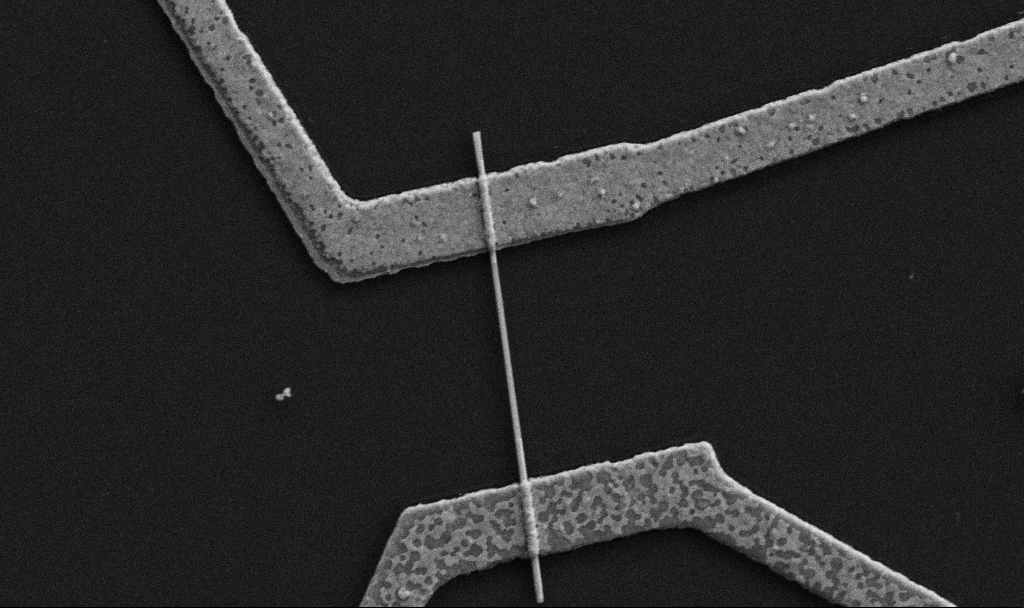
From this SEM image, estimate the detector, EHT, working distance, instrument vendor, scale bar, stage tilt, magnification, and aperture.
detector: SE2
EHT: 5 kV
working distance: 8.7 mm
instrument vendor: Zeiss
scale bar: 1000 nm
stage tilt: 0°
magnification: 30 K X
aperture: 30 µm